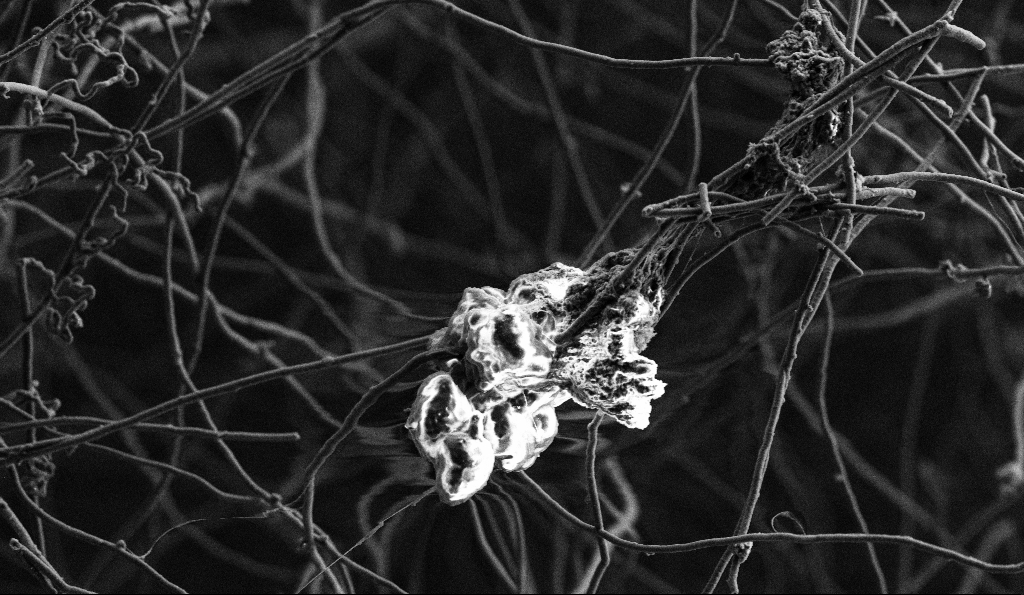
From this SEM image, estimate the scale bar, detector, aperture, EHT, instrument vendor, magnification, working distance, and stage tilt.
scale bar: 10000 nm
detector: SE2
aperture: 30 µm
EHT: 3 kV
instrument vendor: Zeiss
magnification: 5 K X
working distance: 5.5 mm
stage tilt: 0°